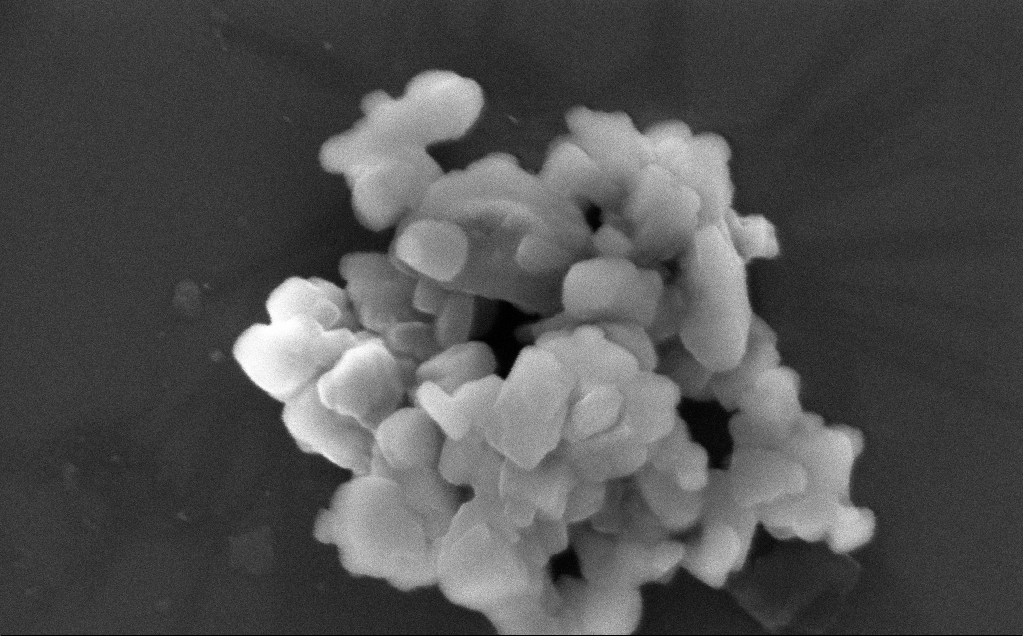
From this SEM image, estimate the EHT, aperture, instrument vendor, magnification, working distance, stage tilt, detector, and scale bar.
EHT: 3 kV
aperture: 30 µm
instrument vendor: Zeiss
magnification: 189.31 K X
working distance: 3 mm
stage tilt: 0°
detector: InLens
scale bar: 200 nm